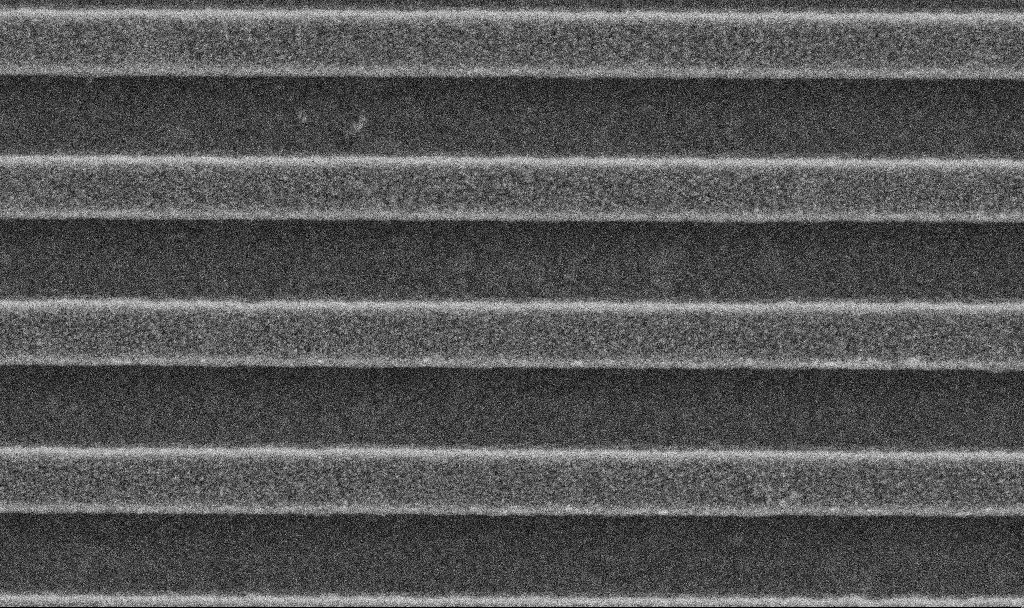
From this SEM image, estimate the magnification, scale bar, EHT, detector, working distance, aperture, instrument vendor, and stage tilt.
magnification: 90.46 K X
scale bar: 200 nm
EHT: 5 kV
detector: SE2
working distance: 2.9 mm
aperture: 30 µm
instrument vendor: Zeiss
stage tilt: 0°